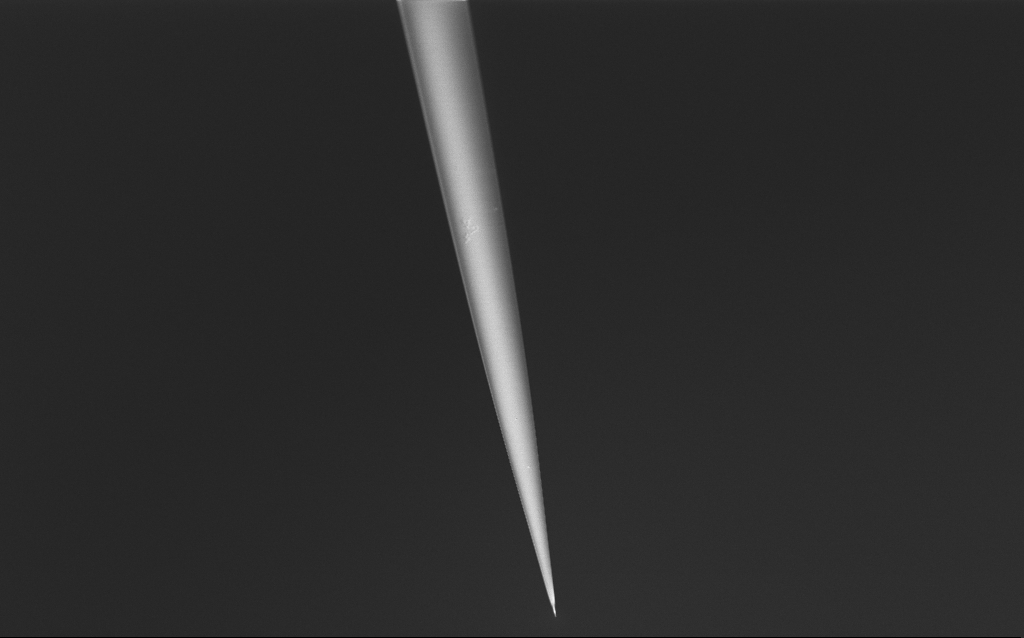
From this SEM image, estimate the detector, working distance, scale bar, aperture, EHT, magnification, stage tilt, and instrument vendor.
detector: InLens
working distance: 6 mm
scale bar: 20000 nm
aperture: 30 µm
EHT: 1.5 kV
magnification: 1 K X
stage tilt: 45°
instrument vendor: Zeiss